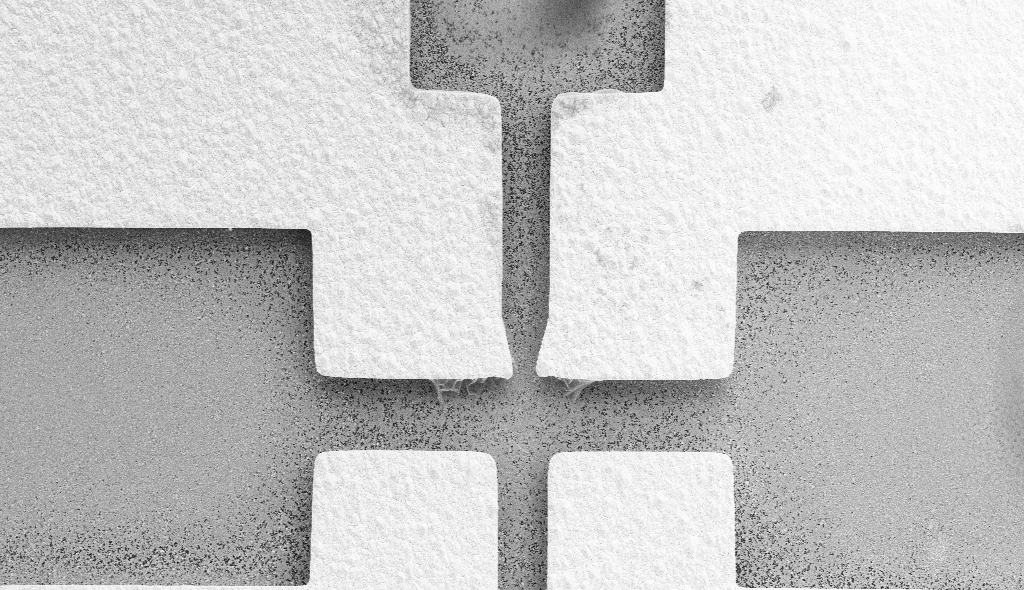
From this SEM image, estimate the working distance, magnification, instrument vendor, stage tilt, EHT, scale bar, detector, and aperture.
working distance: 17 mm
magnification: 1.91 K X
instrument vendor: Zeiss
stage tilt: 0°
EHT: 10 kV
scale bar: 10000 nm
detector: SE2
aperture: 30 µm